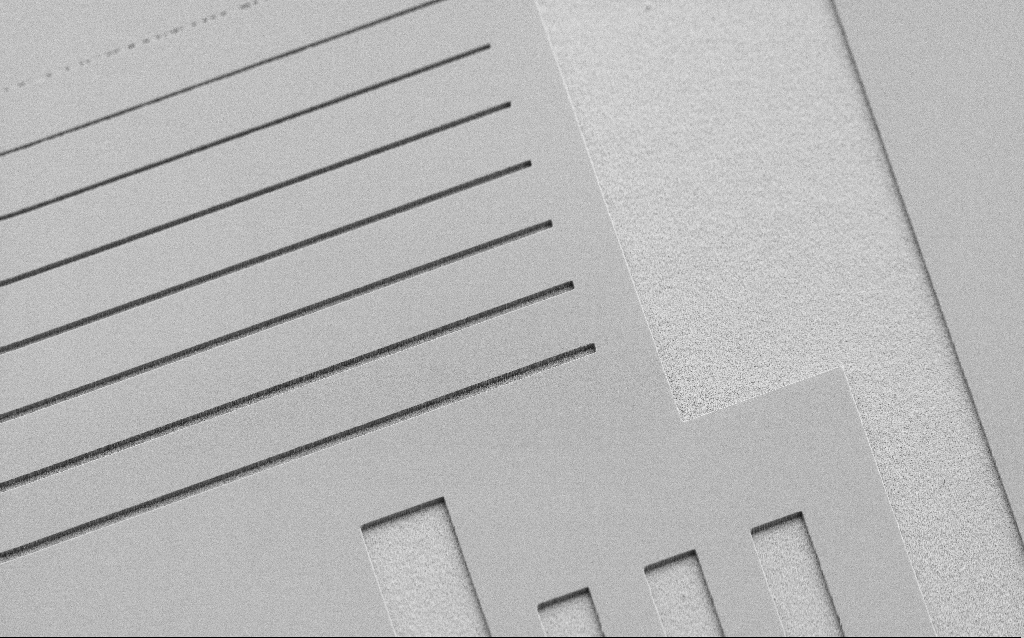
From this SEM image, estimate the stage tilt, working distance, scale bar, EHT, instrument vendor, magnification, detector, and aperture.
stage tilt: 45°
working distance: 7 mm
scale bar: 100000 nm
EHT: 3 kV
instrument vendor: Zeiss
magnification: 0.414 K X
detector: SE2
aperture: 30 µm